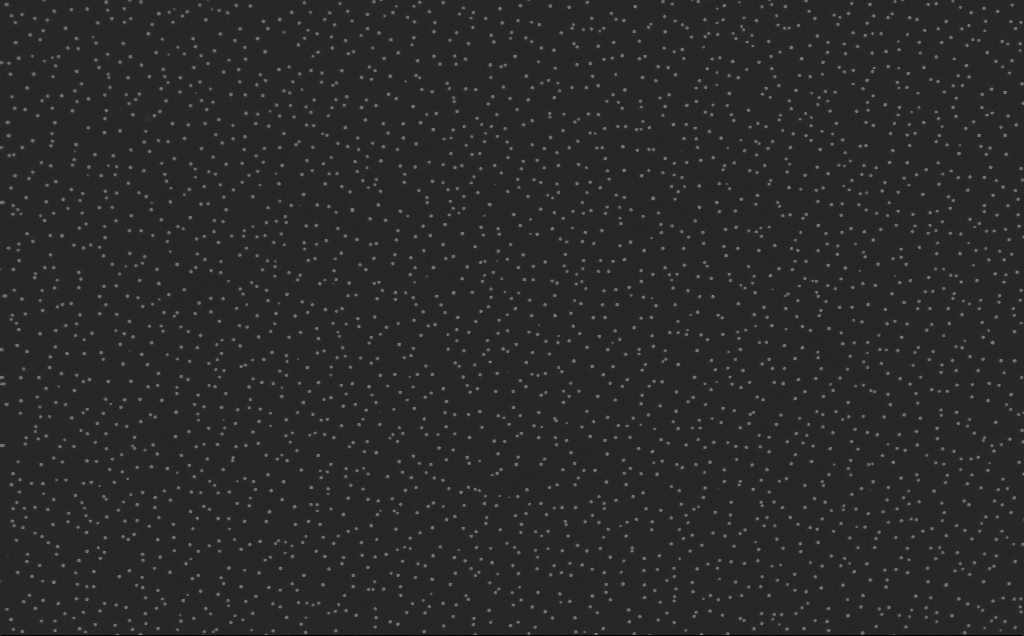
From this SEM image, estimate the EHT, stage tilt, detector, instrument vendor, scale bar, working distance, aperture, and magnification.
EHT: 10 kV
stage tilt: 0°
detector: InLens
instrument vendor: Zeiss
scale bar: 2000 nm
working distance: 8 mm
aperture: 30 µm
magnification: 20 K X